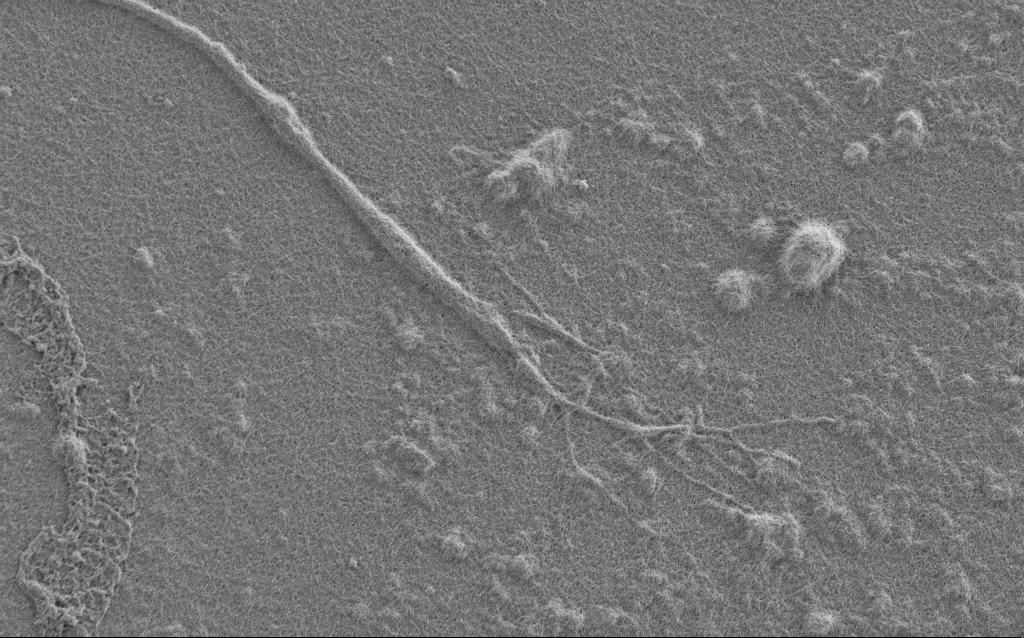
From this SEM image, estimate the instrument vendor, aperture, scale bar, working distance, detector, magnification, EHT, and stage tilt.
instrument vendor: Zeiss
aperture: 30 µm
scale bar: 2000 nm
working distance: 6 mm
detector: SE2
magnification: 7.5 K X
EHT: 1 kV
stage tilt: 0°